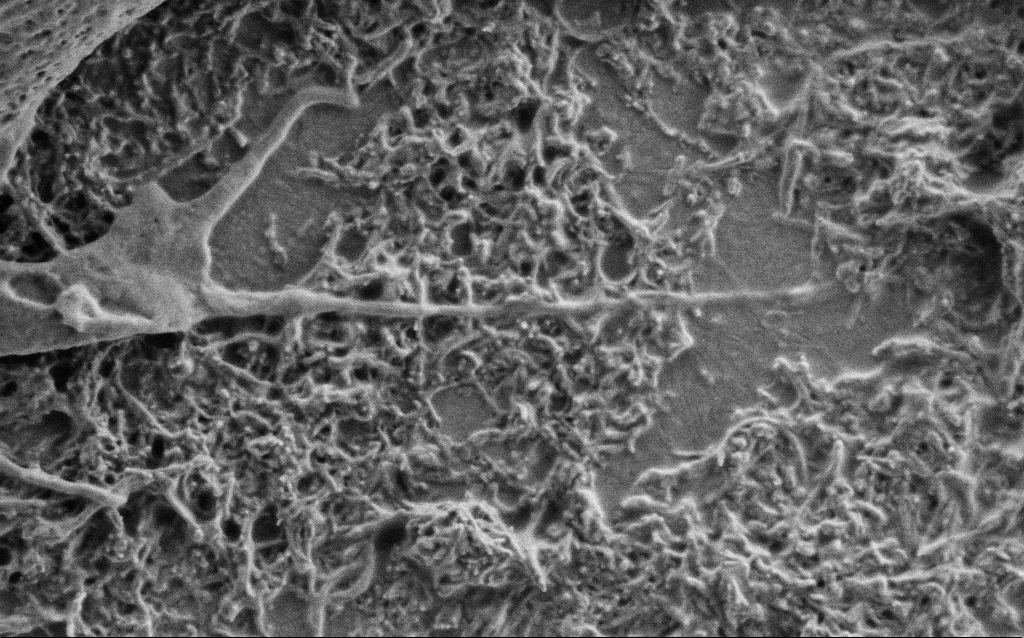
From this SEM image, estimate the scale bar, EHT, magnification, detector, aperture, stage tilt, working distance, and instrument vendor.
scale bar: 1000 nm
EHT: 1 kV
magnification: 50 K X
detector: SE2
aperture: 30 µm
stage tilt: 0°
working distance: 6 mm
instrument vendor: Zeiss